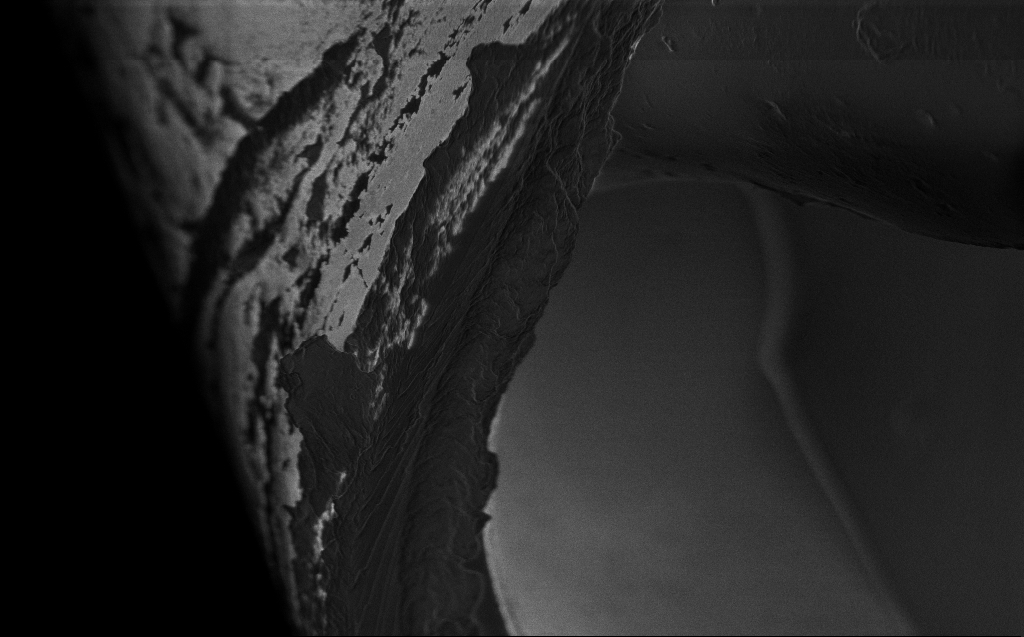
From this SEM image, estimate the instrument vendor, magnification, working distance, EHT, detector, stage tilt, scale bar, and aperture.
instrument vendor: Zeiss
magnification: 10.64 K X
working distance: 3 mm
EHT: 1 kV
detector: InLens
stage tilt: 45°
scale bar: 2000 nm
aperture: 30 µm